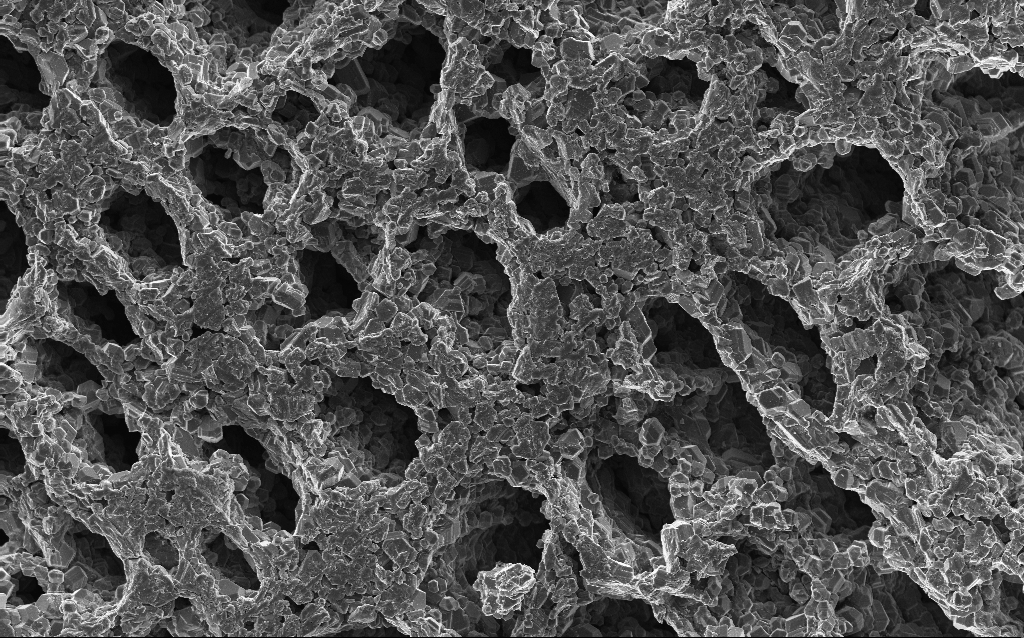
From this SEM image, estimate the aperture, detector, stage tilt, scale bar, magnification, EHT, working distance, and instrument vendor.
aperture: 30 µm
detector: InLens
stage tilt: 0°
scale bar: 20000 nm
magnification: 1 K X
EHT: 10 kV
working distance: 3 mm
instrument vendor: Zeiss